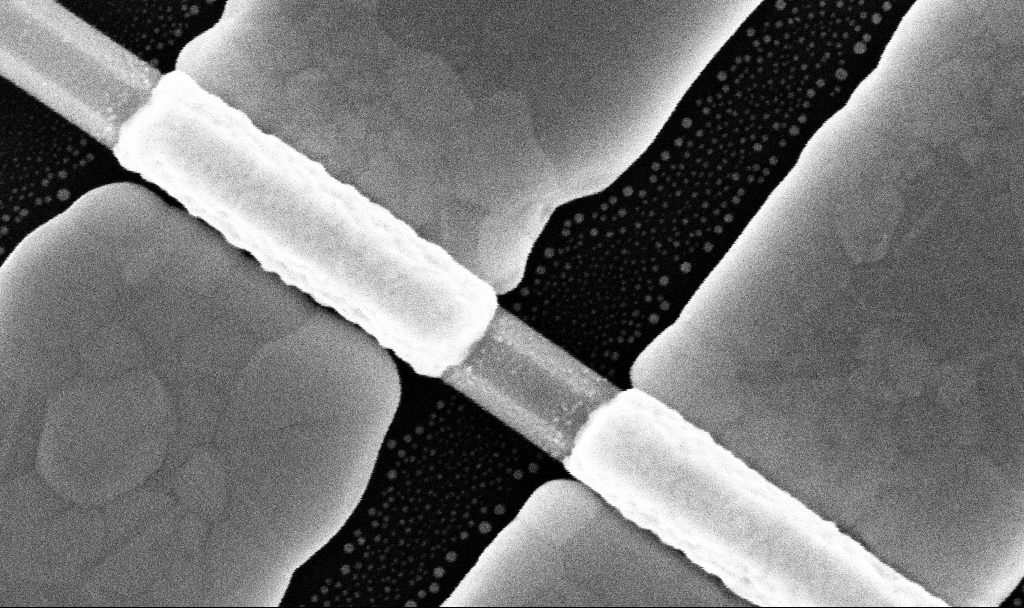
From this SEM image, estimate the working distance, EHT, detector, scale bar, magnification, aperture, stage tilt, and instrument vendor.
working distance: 7.7 mm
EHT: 10 kV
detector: InLens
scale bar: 200 nm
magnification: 231.83 K X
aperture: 30 µm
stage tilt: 0°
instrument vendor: Zeiss